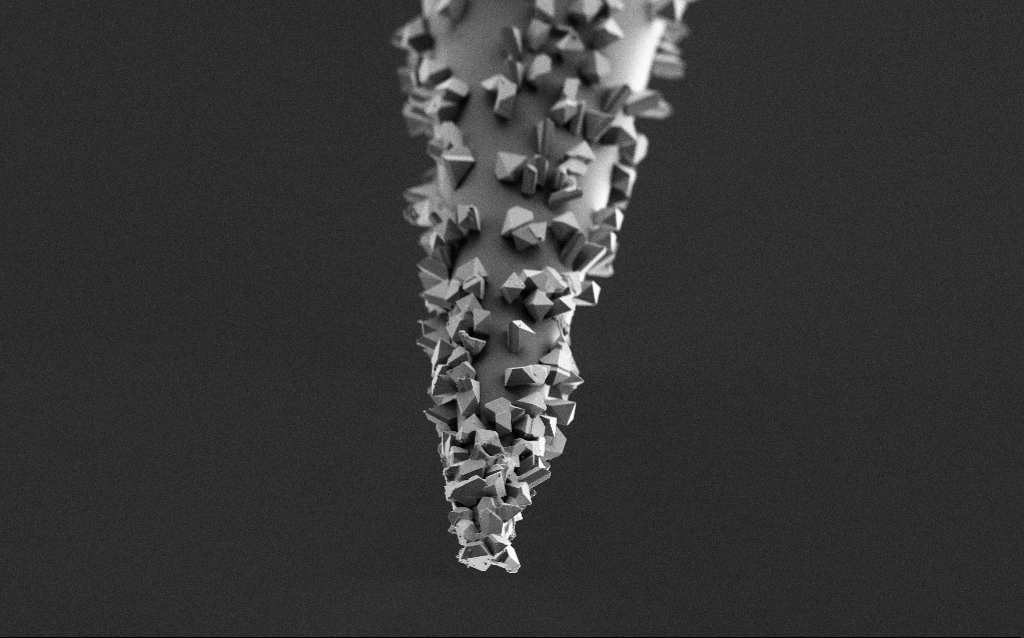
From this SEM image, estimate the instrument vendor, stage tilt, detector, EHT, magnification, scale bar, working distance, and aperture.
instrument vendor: Zeiss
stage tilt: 45°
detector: SE2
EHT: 2 kV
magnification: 5 K X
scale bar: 10000 nm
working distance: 4 mm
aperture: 30 µm